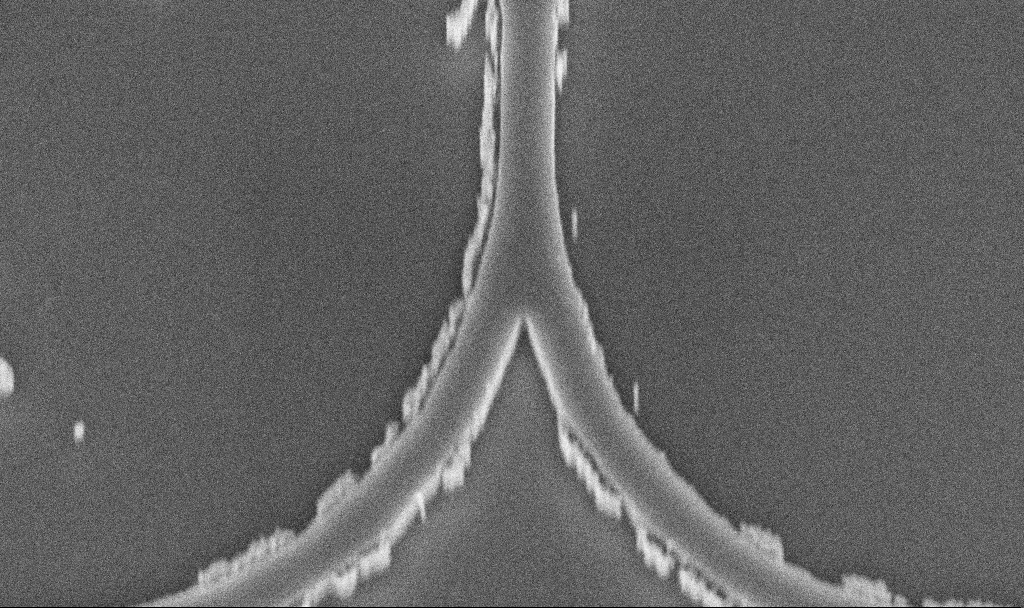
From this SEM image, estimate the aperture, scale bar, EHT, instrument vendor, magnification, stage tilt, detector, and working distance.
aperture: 30 µm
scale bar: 1000 nm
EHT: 5 kV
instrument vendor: Zeiss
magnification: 38.3 K X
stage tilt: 45°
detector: InLens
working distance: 9.5 mm